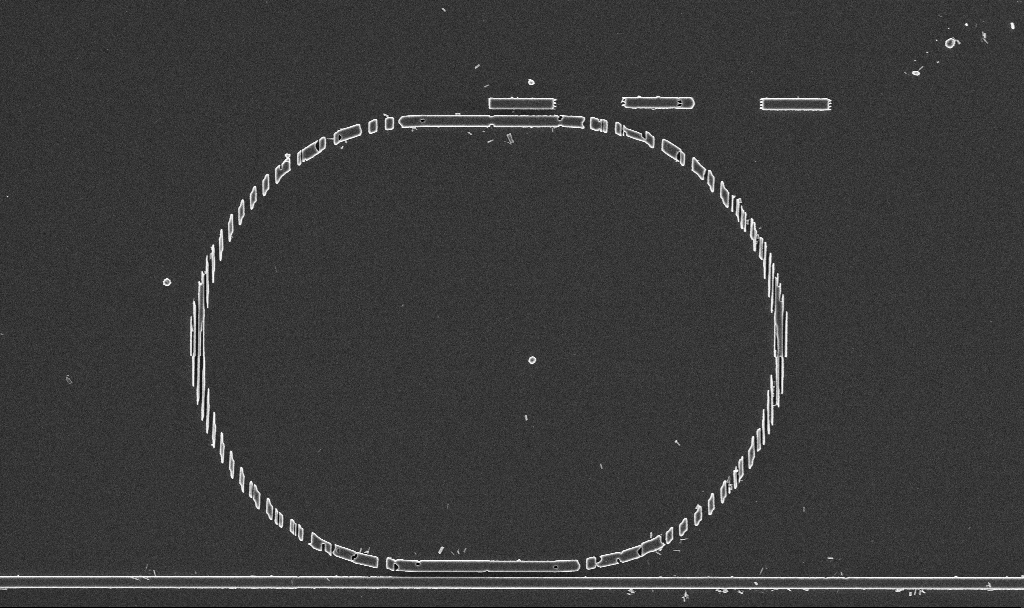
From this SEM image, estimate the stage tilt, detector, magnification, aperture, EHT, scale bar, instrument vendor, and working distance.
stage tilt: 0°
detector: InLens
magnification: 8.53 K X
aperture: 30 µm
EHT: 3 kV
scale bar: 2000 nm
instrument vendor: Zeiss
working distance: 2.9 mm